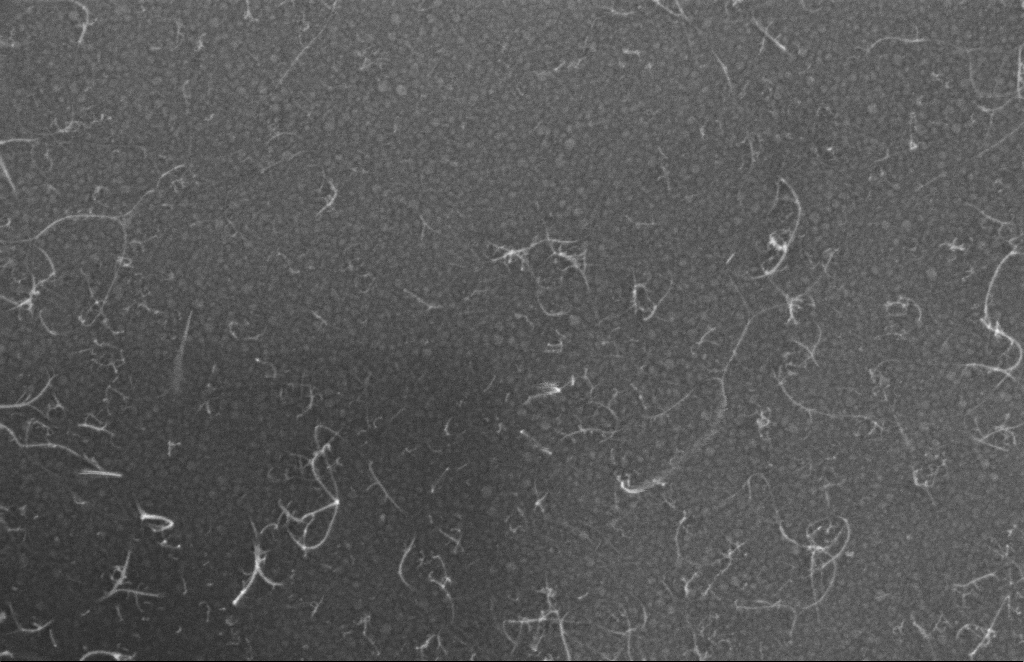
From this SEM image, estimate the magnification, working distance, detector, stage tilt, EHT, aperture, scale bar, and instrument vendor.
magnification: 175.33 K X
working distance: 4 mm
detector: InLens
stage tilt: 0°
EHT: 5 kV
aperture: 20 µm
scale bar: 100 nm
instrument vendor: Zeiss